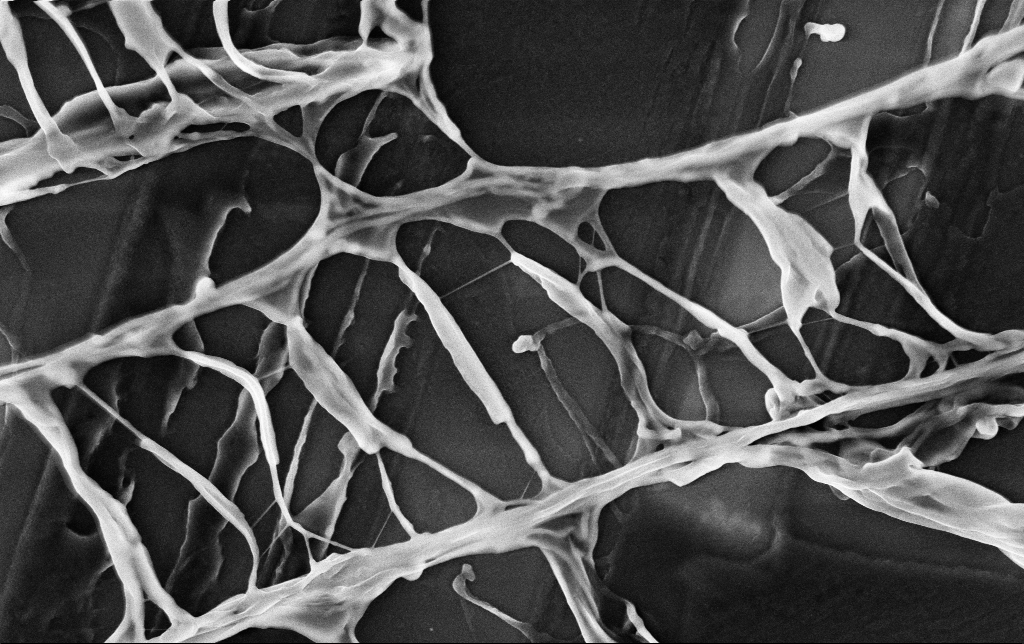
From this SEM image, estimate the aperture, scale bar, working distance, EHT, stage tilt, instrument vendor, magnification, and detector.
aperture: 30 µm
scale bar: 2000 nm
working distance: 3.5 mm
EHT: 3 kV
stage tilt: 0°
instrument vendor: Zeiss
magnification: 30.93 K X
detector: InLens